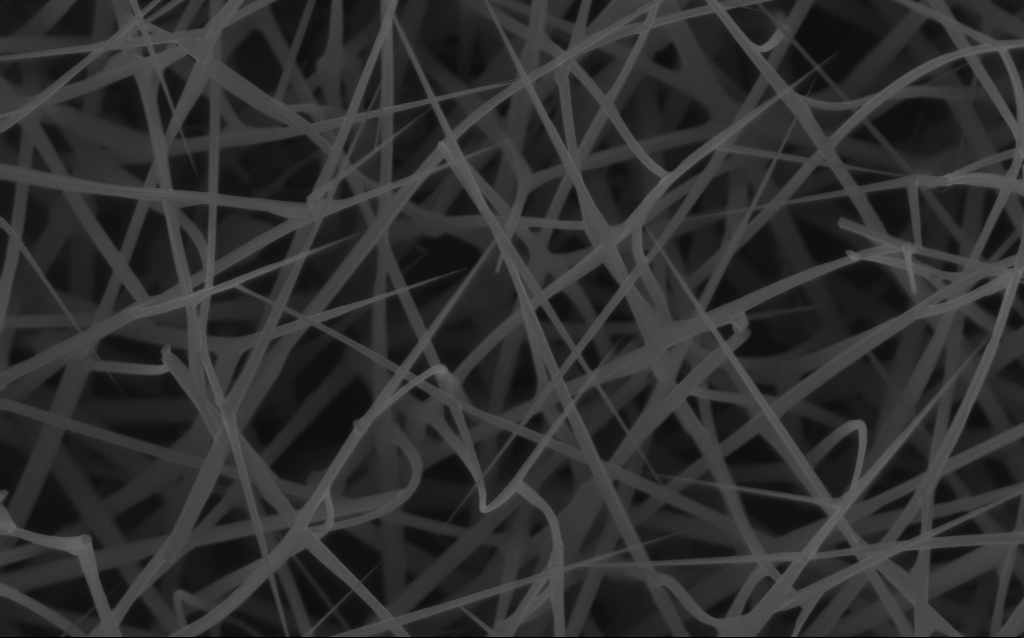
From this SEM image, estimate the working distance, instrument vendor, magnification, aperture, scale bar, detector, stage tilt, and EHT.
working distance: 6 mm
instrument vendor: Zeiss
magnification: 40 K X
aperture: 30 µm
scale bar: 1000 nm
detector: InLens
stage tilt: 0°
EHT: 10 kV